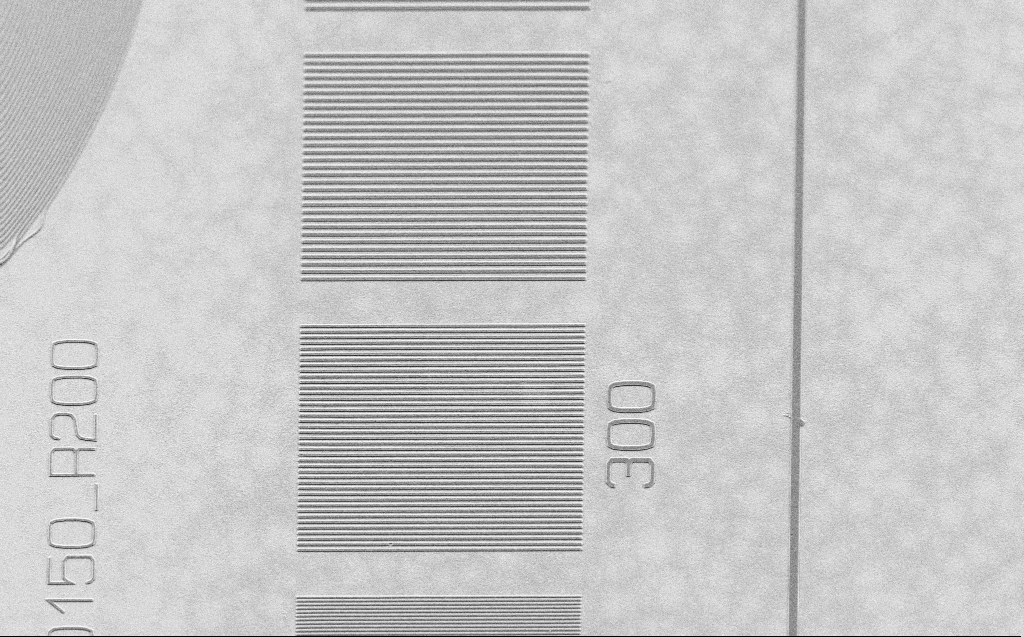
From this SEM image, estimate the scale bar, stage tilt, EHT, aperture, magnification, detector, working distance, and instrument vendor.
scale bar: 10000 nm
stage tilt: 30°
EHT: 2.5 kV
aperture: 30 µm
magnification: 3.51 K X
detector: SE2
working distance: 5 mm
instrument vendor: Zeiss